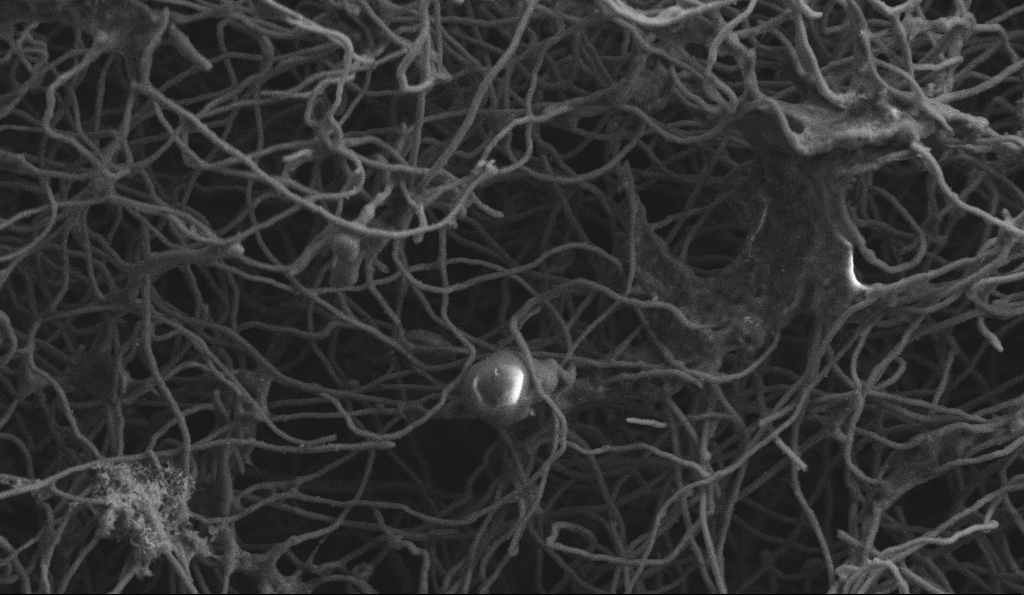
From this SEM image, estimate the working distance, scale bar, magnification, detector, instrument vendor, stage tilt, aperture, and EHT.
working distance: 5 mm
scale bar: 2000 nm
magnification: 15 K X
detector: SE2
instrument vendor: Zeiss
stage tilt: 0°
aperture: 30 µm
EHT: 3 kV